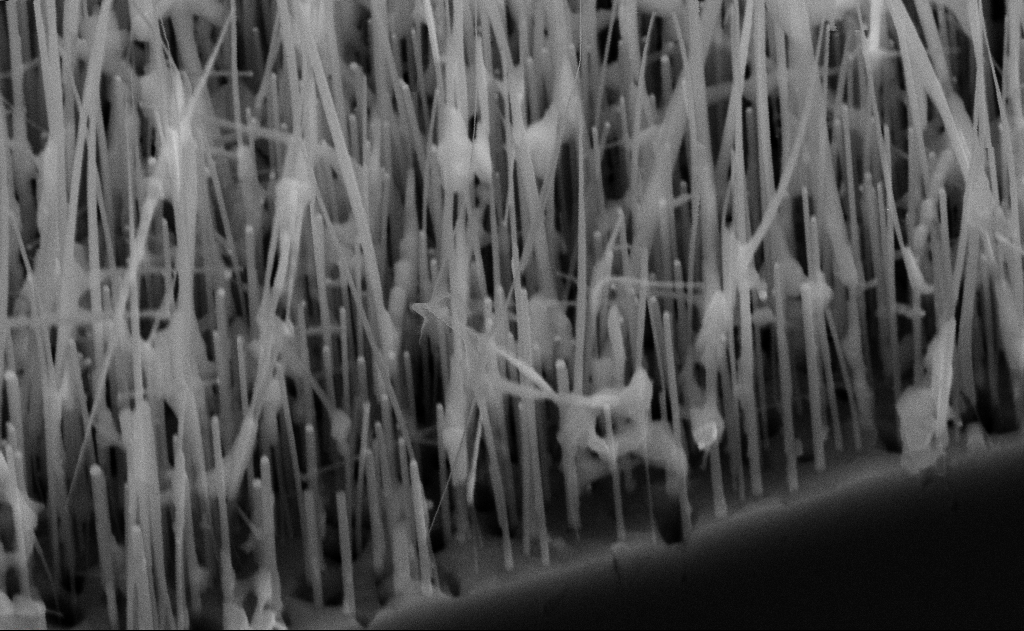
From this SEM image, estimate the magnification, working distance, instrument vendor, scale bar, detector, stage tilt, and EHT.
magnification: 80 K X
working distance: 12 mm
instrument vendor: Zeiss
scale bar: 200 nm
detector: SE2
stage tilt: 45°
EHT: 10 kV